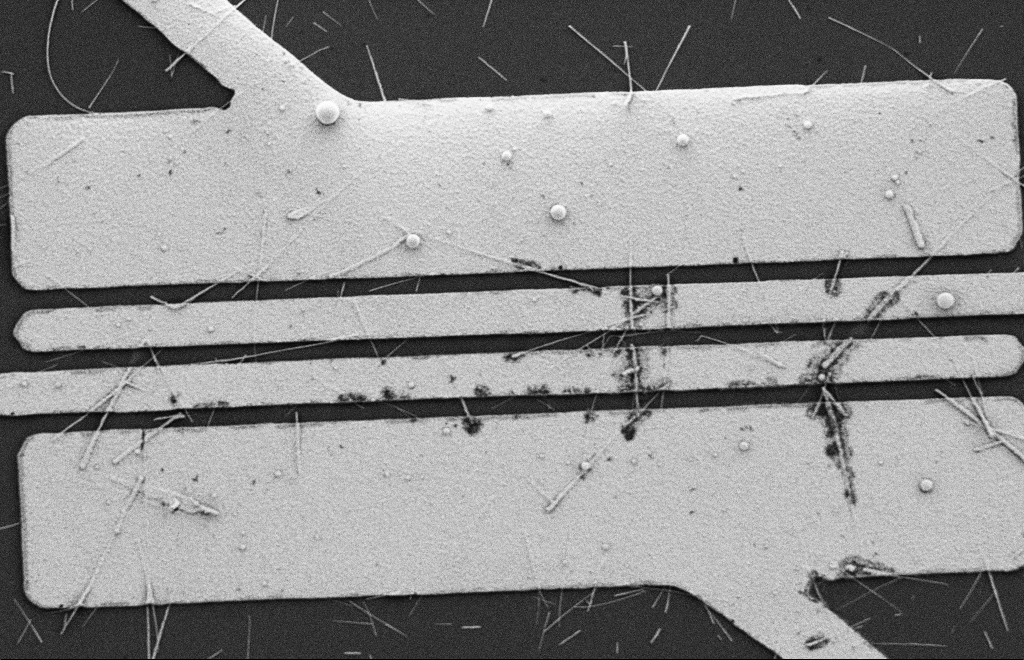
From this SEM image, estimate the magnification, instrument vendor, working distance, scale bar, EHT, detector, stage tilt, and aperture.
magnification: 6.04 K X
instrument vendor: Zeiss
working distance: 9 mm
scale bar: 2000 nm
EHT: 2 kV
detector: SE2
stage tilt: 0°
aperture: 20 µm